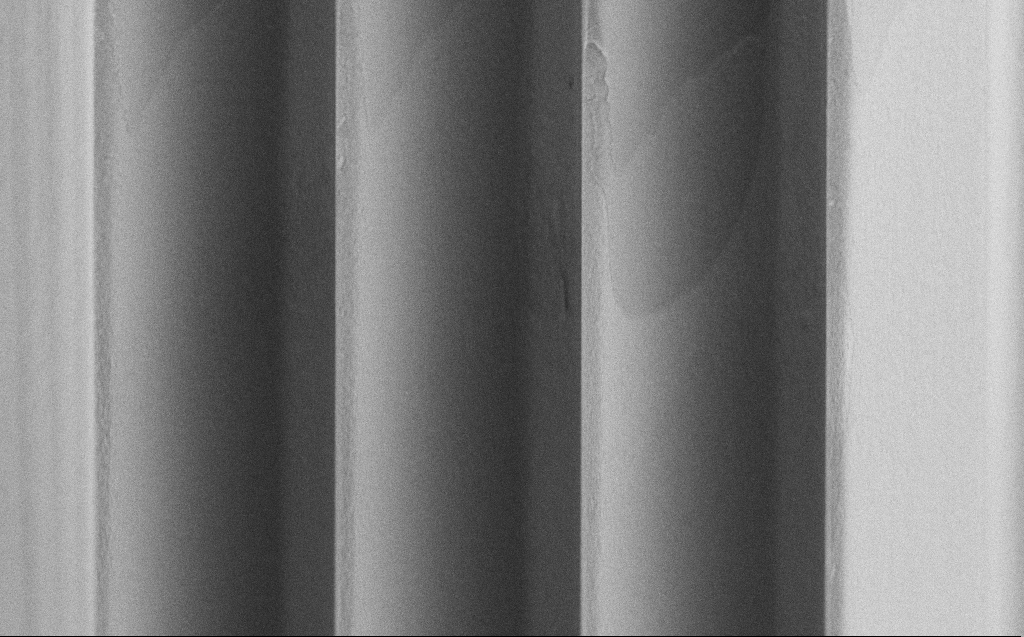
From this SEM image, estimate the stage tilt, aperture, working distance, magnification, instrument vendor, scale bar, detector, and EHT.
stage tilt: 45°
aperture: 30 µm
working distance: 5 mm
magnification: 7.91 K X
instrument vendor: Zeiss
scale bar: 2000 nm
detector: SE2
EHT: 5 kV